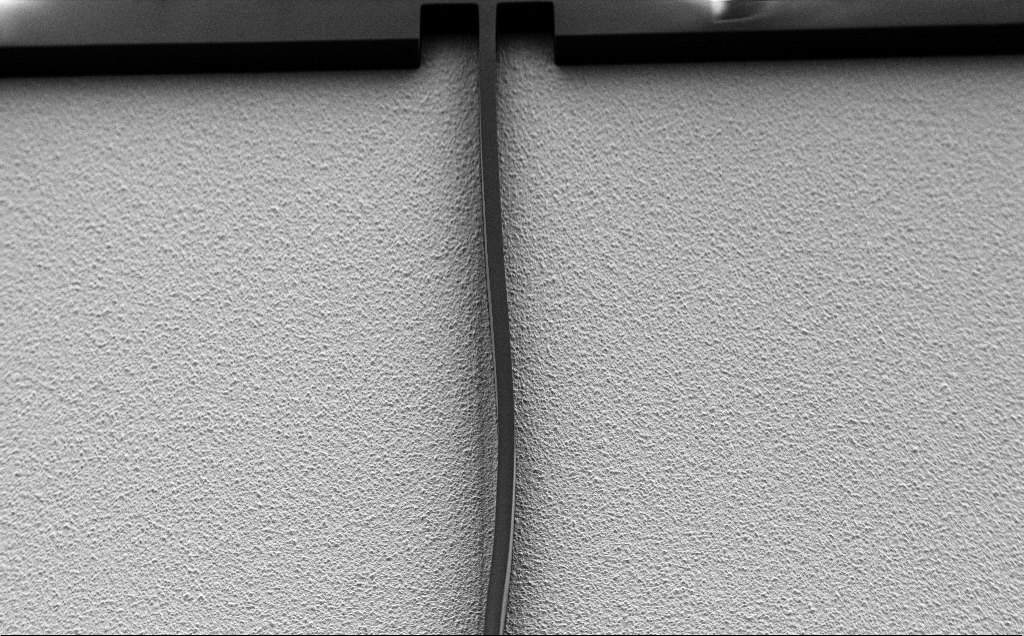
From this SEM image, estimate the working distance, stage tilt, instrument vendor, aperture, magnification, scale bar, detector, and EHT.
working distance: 7 mm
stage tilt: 37.9°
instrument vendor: Zeiss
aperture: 30 µm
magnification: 0.613 K X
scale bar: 100000 nm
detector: SE2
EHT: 1.5 kV